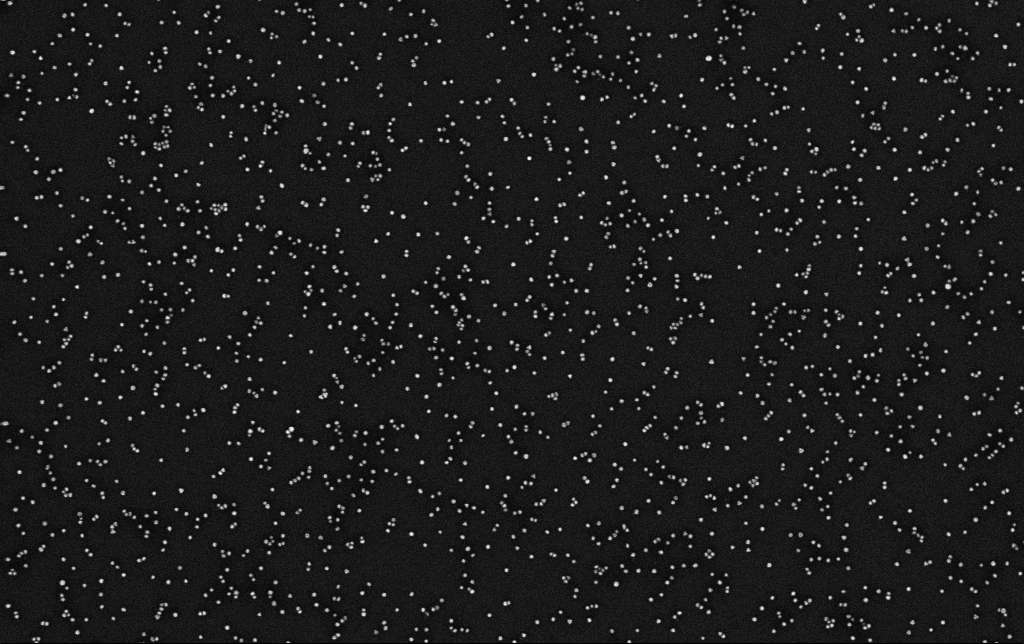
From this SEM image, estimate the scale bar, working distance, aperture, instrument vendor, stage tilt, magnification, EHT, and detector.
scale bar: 200 nm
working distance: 3.3 mm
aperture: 30 µm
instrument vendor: Zeiss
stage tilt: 0°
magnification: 100 K X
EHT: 10 kV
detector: InLens